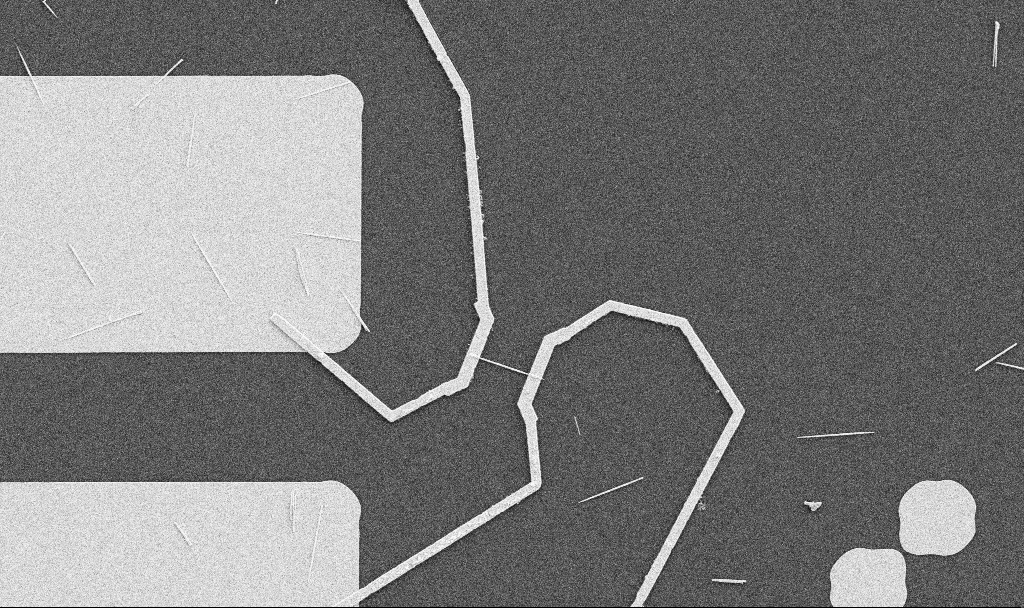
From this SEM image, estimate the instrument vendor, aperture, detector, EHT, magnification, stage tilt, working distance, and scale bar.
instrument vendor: Zeiss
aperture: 30 µm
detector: SE2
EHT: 5 kV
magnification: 5 K X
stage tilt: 0°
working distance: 10.7 mm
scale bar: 10000 nm